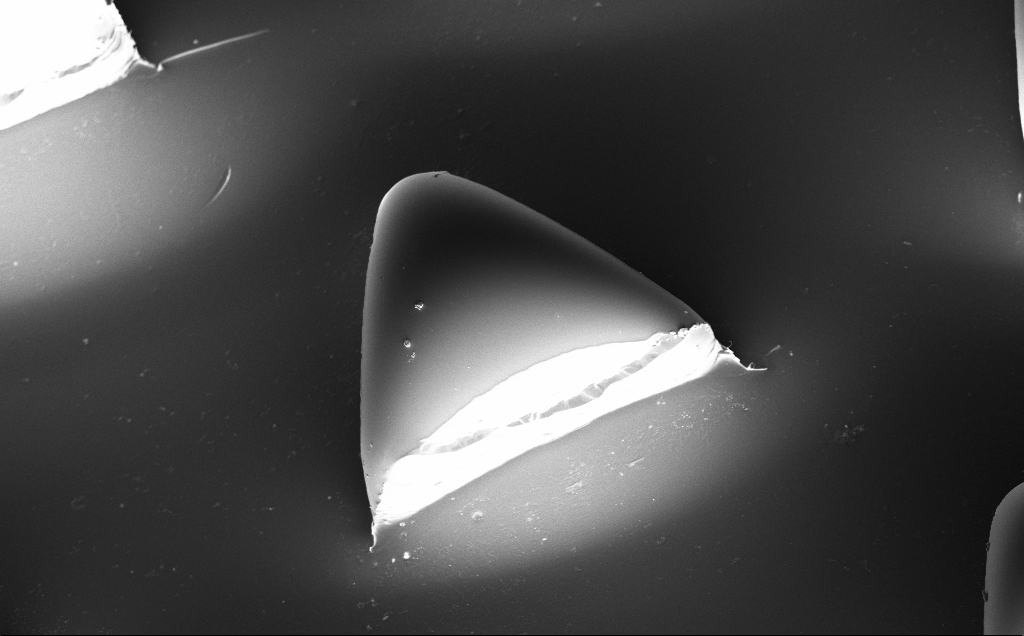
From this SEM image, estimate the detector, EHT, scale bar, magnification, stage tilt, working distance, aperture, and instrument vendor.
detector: InLens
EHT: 10 kV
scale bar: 100000 nm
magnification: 0.604 K X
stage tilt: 20°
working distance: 10 mm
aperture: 30 µm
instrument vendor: Zeiss